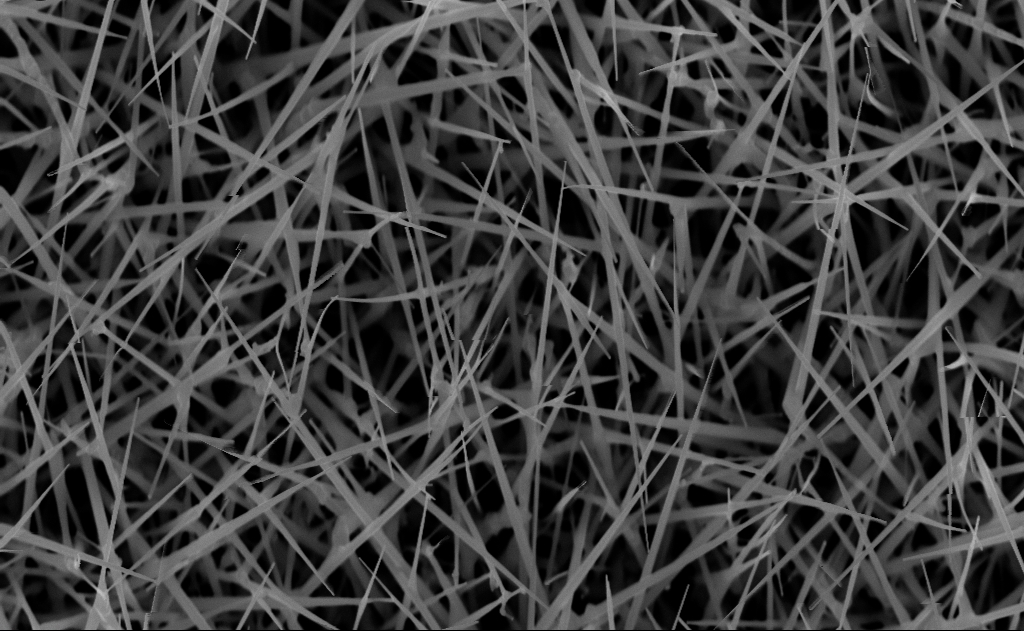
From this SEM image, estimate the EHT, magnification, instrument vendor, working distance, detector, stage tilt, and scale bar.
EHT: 10 kV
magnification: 40 K X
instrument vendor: Zeiss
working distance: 7 mm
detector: InLens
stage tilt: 0°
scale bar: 1000 nm